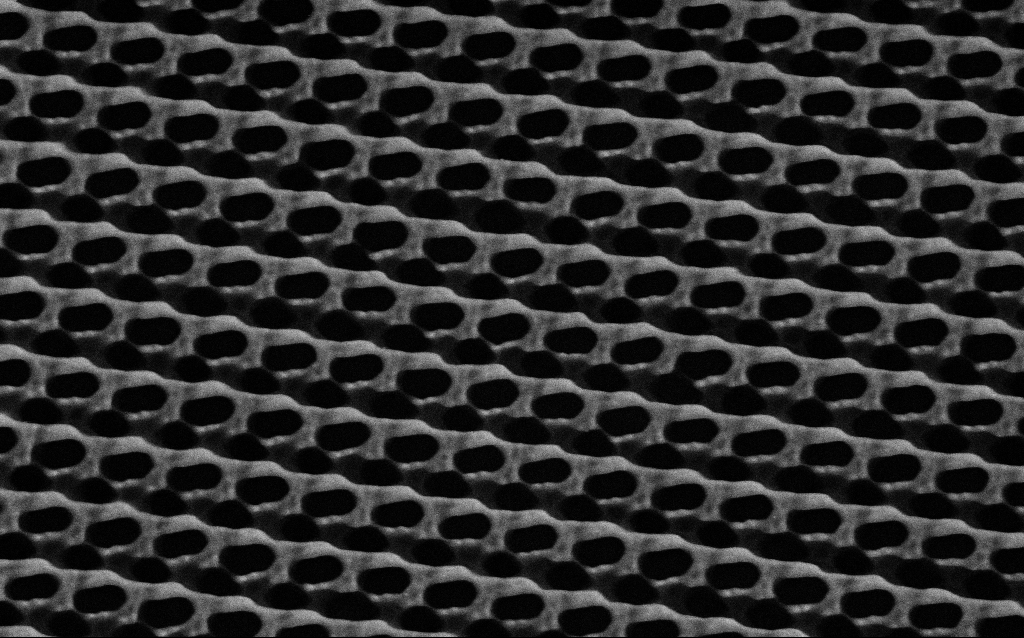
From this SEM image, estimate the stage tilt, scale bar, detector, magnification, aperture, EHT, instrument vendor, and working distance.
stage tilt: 0°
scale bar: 1000 nm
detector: SE2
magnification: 54.92 K X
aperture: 30 µm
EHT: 3 kV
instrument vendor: Zeiss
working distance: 4.6 mm